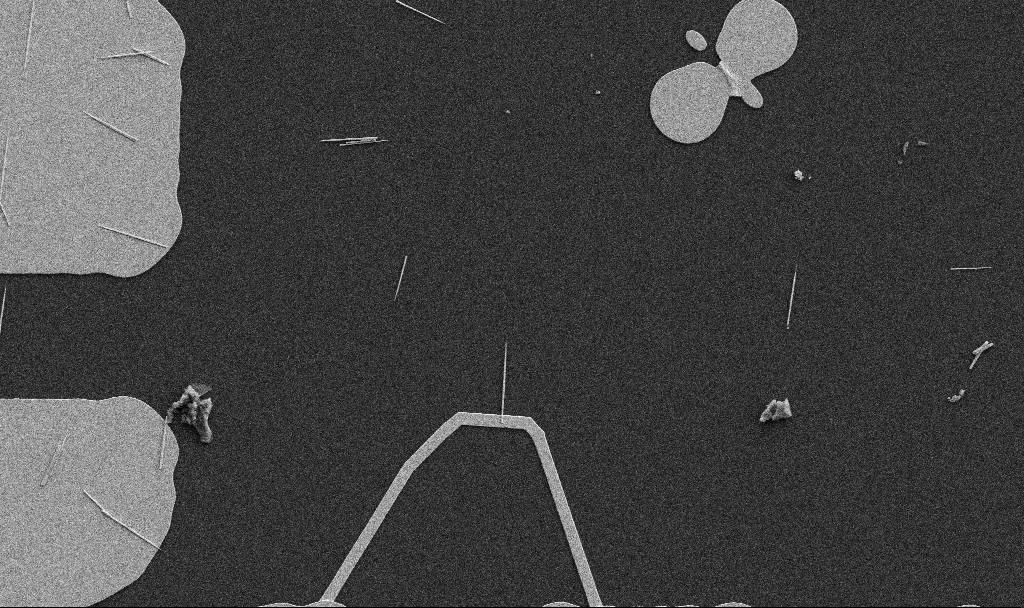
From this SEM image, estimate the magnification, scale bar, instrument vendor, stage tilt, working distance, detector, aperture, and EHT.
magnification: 5 K X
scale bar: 10000 nm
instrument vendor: Zeiss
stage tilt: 0°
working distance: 10.7 mm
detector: SE2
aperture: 30 µm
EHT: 5 kV